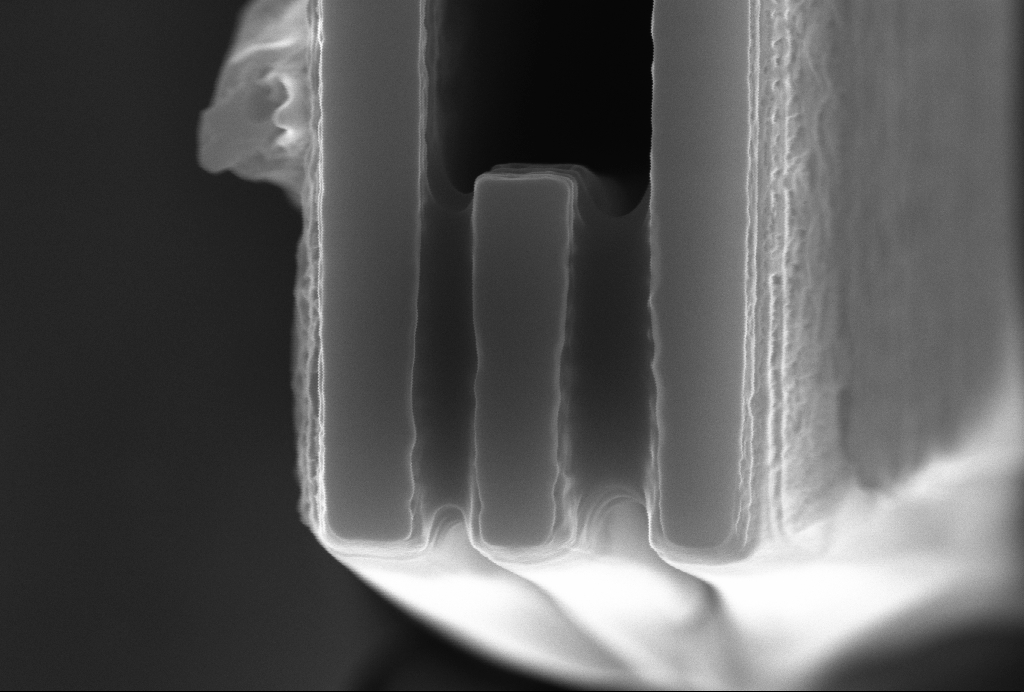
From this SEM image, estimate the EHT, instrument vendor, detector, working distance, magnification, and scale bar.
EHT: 10 kV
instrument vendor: Zeiss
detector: InLens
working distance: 5 mm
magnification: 74.06 K X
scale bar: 200 nm